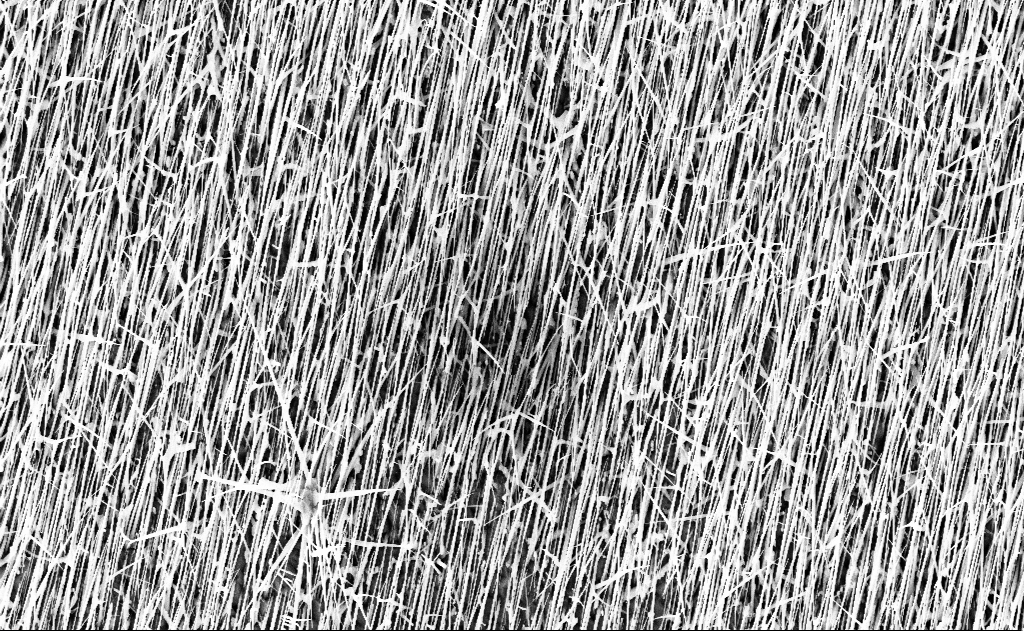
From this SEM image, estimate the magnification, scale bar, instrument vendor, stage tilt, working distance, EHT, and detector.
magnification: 10 K X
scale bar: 2000 nm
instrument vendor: Zeiss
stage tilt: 0°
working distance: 16 mm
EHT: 10 kV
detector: InLens